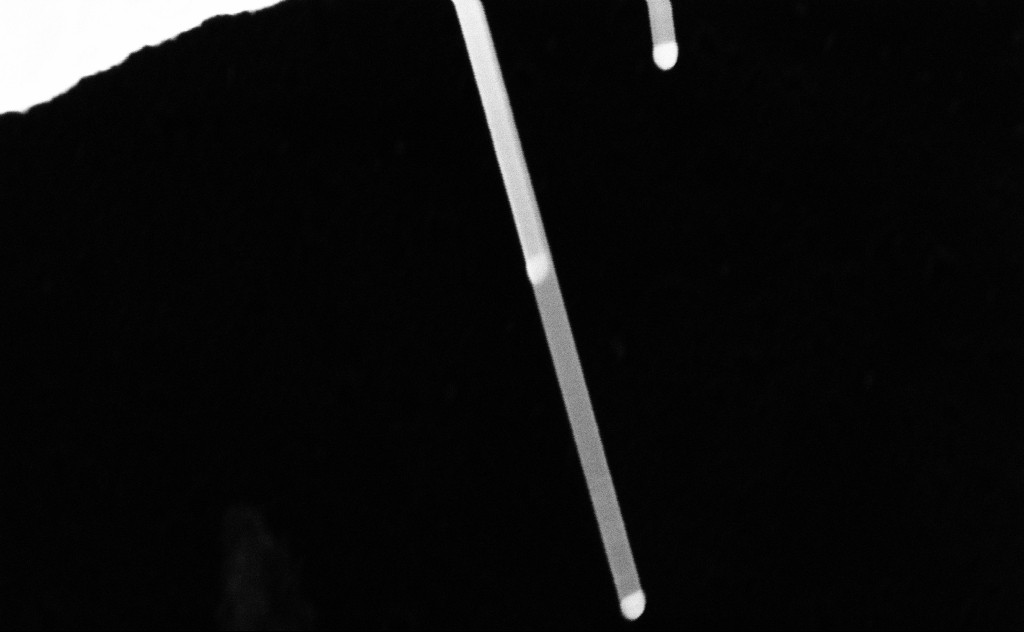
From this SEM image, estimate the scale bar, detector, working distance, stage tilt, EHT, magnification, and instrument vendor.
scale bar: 200 nm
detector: InLens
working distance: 8 mm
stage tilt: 0°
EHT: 20 kV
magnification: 145.21 K X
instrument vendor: Zeiss